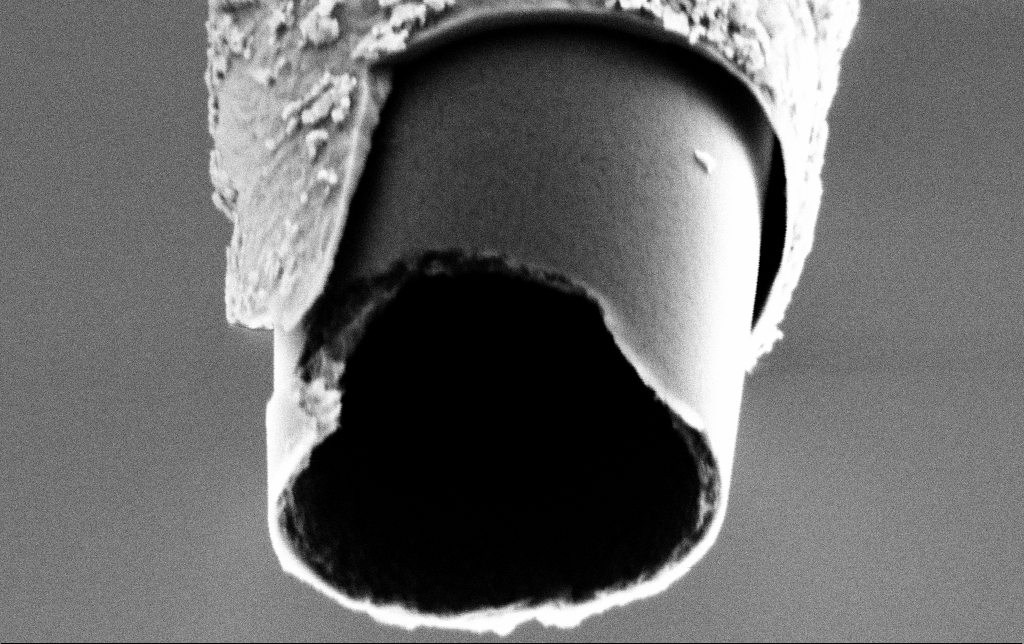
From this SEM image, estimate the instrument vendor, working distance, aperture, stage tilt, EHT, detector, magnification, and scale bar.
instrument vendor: Zeiss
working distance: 7.6 mm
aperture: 30 µm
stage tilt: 45°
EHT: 2 kV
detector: SE2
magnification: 75 K X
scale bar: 200 nm